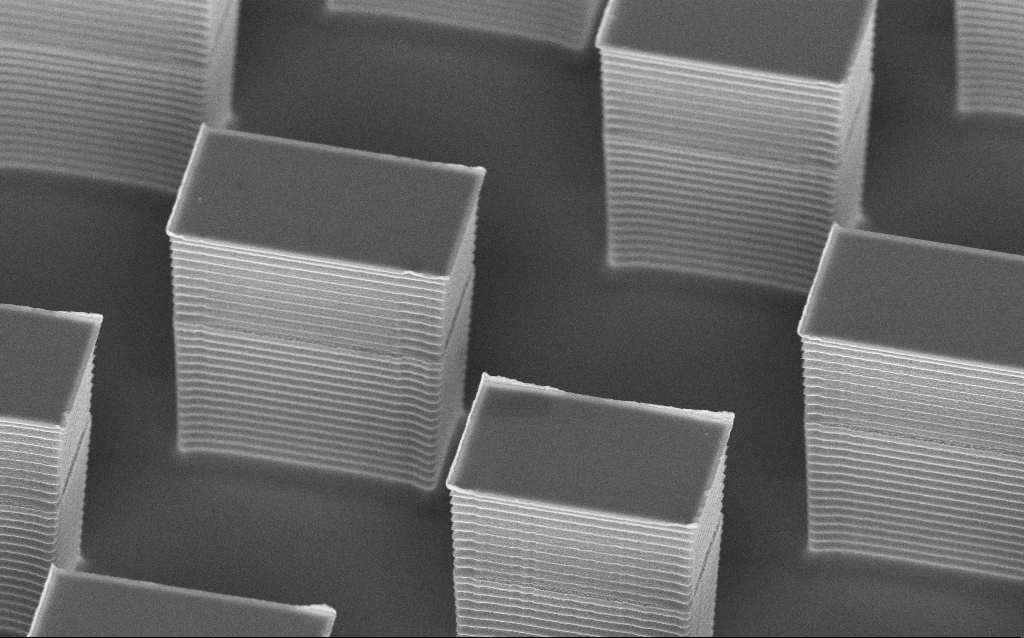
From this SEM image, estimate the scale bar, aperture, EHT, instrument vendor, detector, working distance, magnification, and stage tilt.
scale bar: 2000 nm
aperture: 30 µm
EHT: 5 kV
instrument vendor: Zeiss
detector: InLens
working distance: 8 mm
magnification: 11.83 K X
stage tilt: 45°